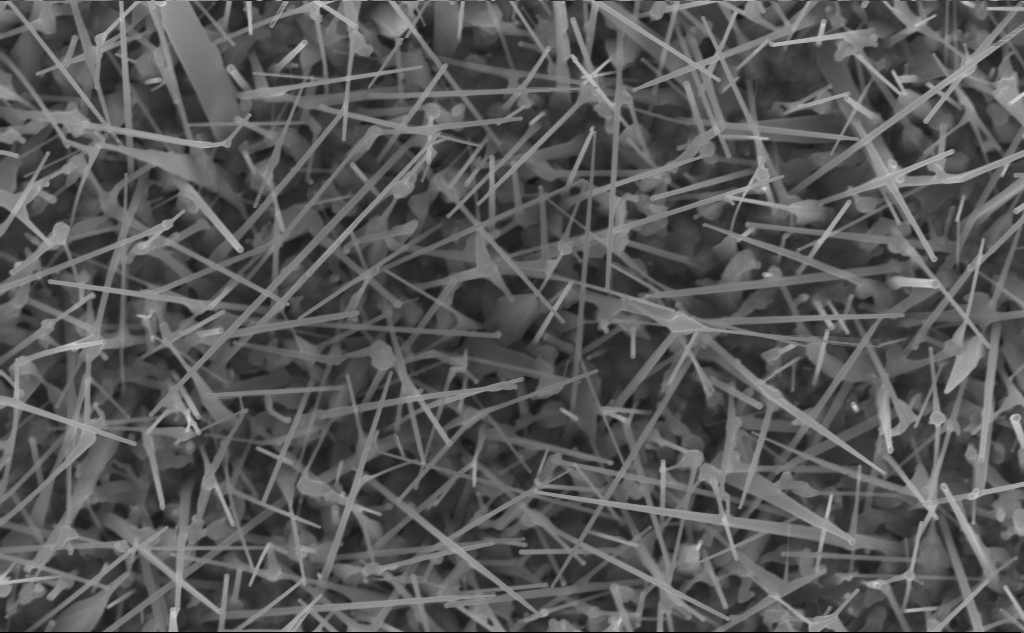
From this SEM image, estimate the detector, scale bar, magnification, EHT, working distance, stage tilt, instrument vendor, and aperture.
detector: InLens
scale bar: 1000 nm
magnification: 40 K X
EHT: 10 kV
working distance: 7 mm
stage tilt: -0.2°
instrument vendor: Zeiss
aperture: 30 µm